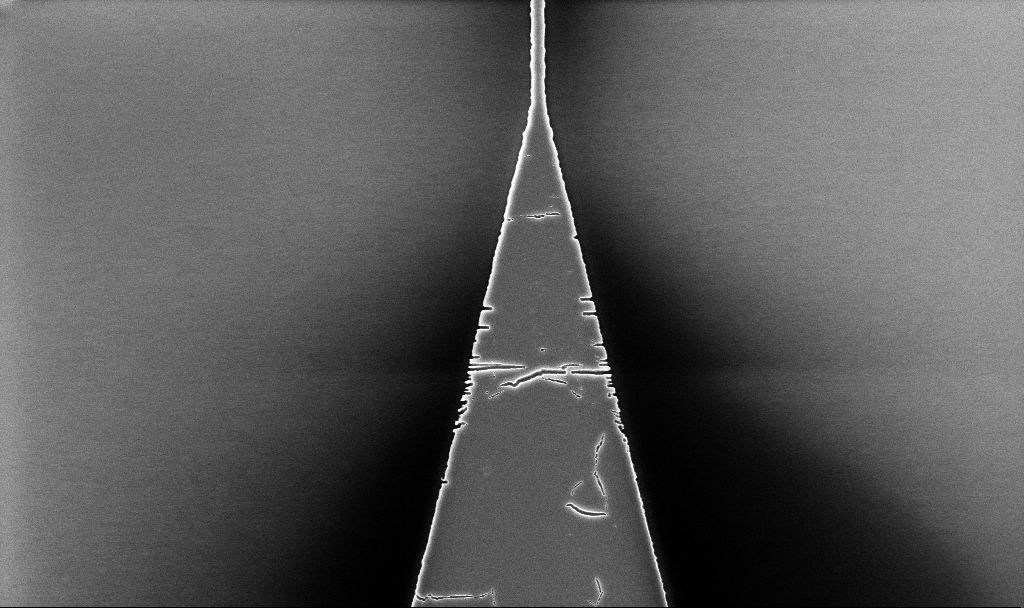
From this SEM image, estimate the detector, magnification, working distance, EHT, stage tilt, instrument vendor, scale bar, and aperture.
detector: InLens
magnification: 9.84 K X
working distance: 5.2 mm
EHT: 5 kV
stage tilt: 0°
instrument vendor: Zeiss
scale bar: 2000 nm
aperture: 30 µm